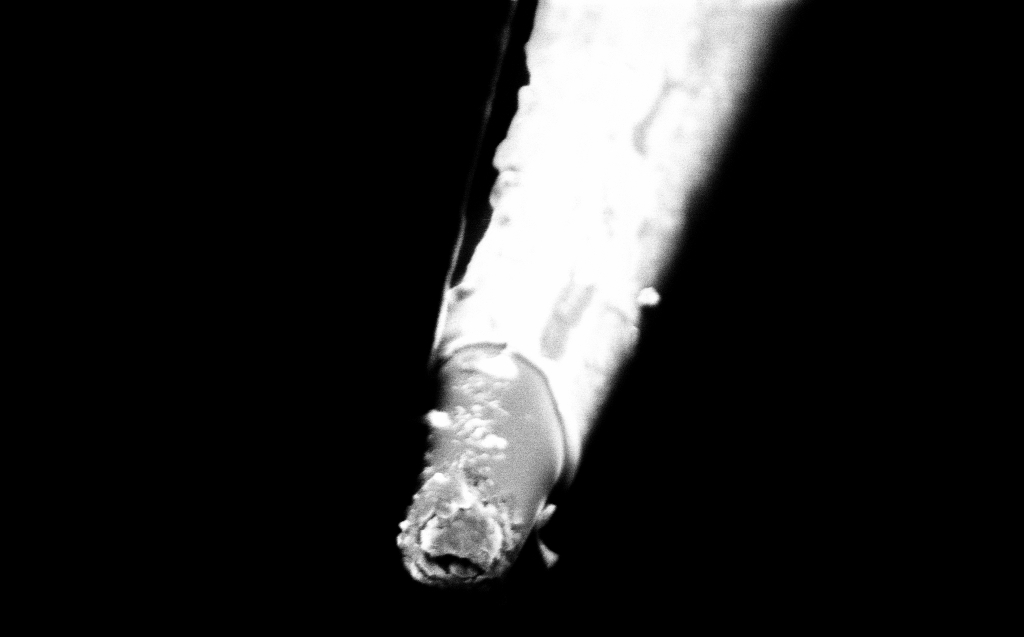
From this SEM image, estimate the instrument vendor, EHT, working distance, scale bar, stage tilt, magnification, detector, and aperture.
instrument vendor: Zeiss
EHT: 2 kV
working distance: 5 mm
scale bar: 1000 nm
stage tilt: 45°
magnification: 50 K X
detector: InLens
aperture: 30 µm